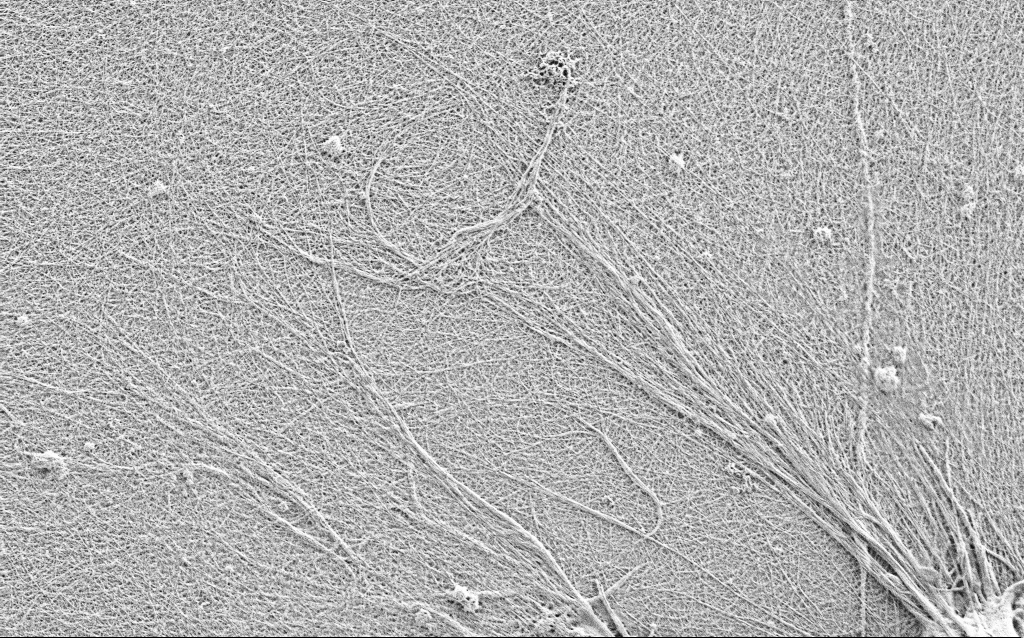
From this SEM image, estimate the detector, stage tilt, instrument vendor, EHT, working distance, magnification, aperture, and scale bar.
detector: SE2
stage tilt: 0°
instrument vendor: Zeiss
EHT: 0.9 kV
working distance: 3 mm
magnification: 10 K X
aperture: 30 µm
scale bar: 2000 nm